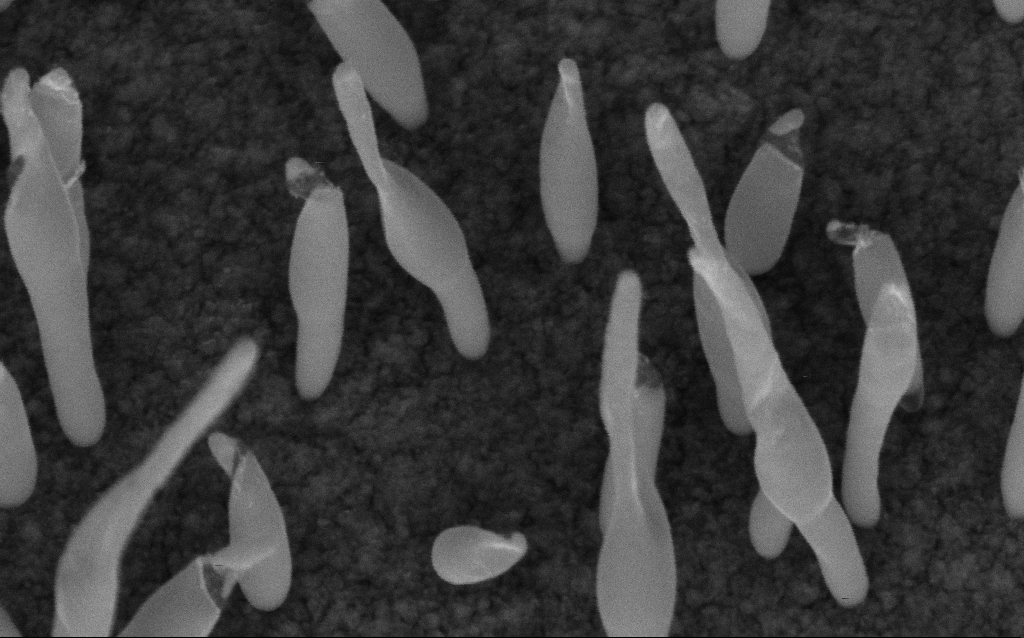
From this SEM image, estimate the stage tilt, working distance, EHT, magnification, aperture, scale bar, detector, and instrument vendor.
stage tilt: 45°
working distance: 7 mm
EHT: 5 kV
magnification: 200 K X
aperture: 30 µm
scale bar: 100 nm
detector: InLens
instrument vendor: Zeiss